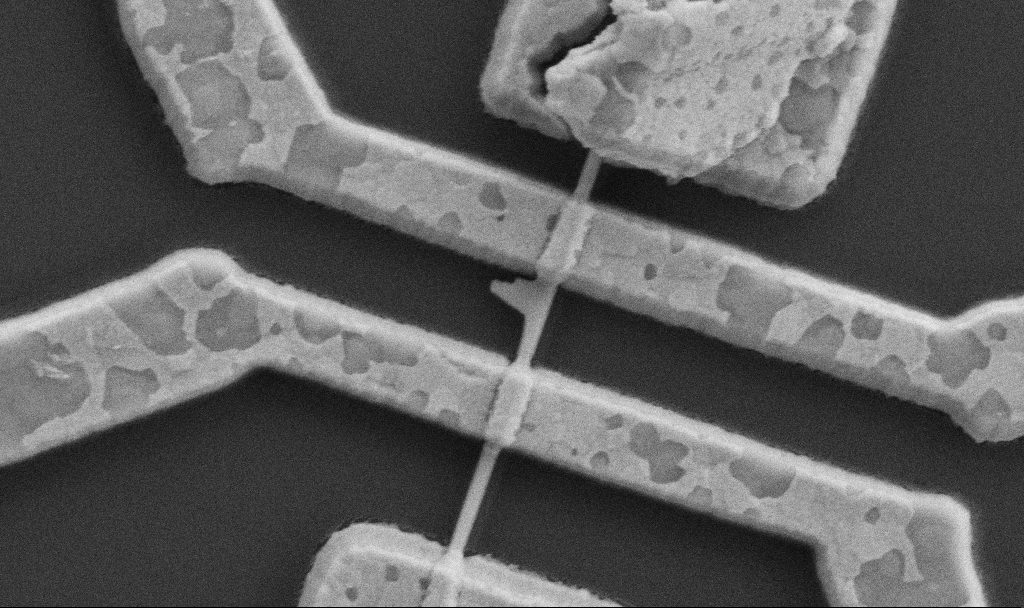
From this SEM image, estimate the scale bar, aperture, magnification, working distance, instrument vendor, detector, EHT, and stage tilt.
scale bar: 1000 nm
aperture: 30 µm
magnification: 60 K X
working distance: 8.7 mm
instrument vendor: Zeiss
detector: SE2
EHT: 5 kV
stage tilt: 0°